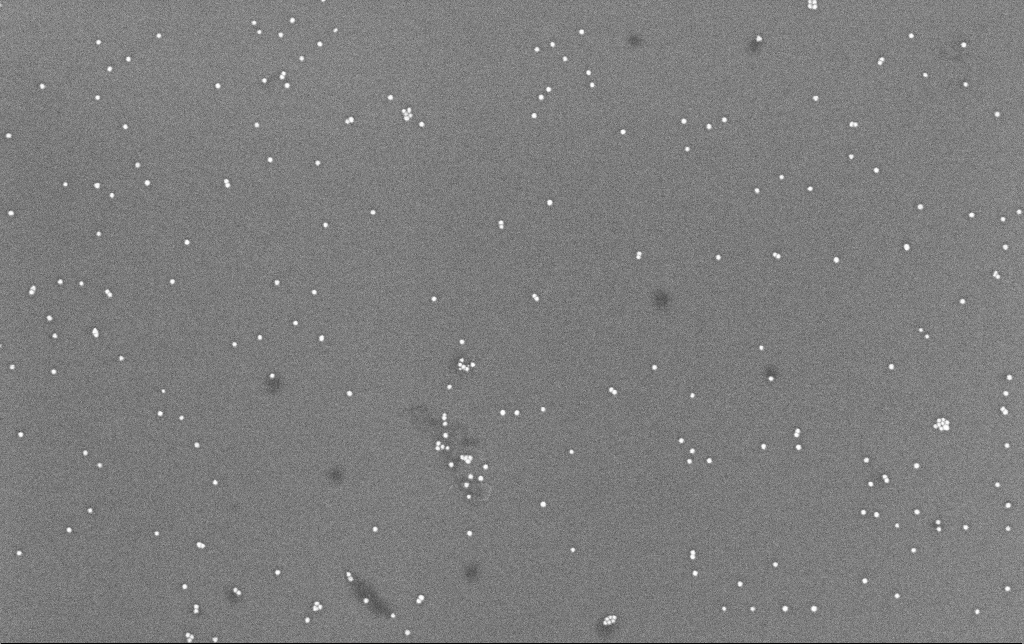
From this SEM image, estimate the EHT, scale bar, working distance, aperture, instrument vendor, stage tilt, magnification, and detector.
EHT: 8 kV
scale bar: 200 nm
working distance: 6.6 mm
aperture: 30 µm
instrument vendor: Zeiss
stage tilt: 0°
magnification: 100 K X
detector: InLens